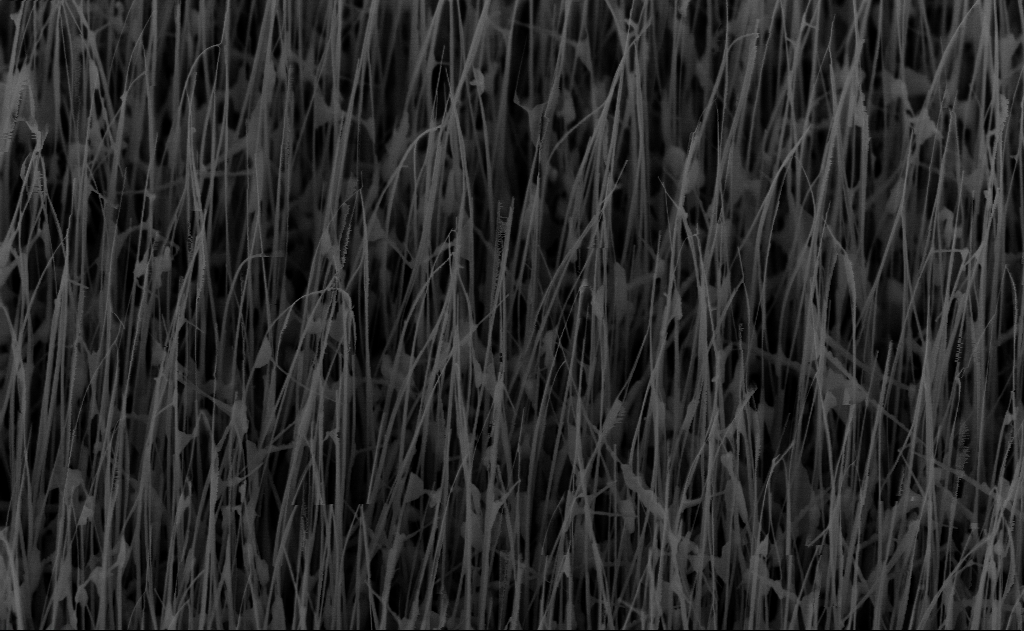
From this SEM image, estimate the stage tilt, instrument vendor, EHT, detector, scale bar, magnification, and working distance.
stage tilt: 45°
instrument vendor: Zeiss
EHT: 10 kV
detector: InLens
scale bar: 1000 nm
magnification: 40 K X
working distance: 7 mm